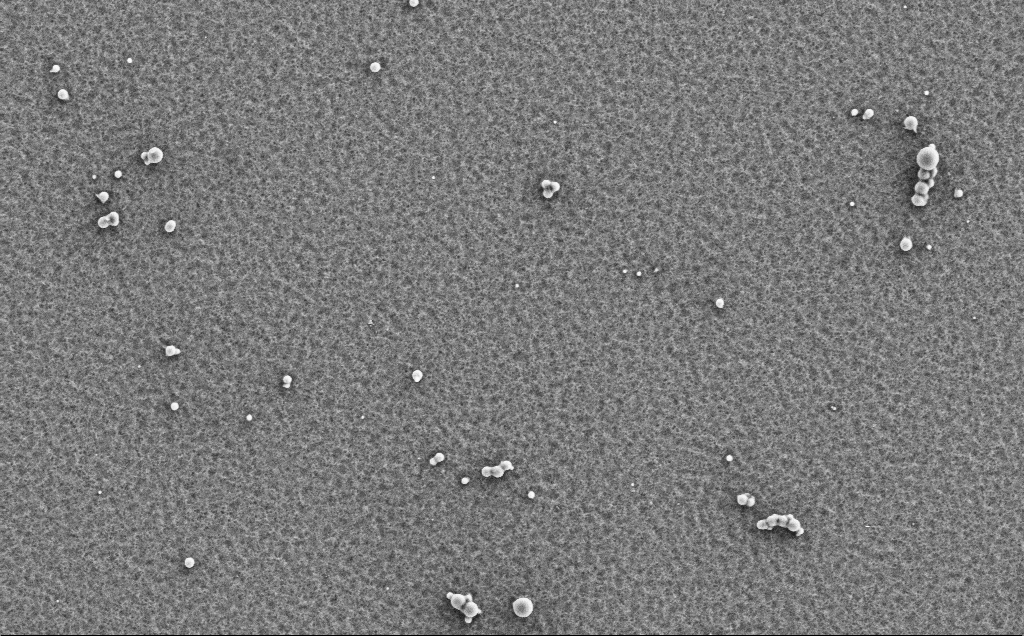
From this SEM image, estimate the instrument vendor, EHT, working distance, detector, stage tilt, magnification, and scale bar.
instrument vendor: Zeiss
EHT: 5 kV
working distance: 5 mm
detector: SE2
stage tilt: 0°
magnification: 4.14 K X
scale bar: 10000 nm